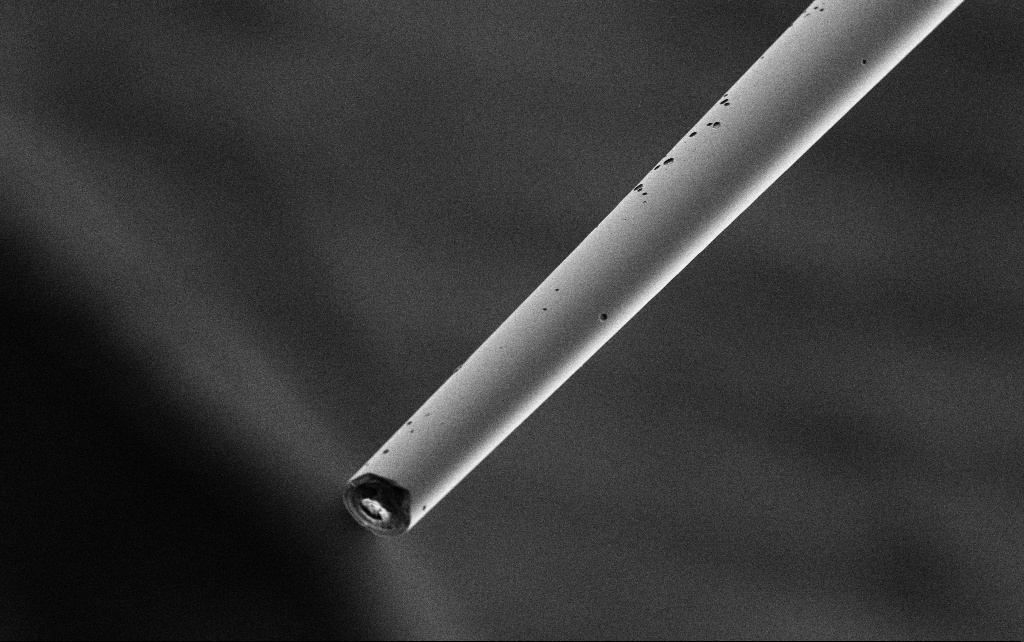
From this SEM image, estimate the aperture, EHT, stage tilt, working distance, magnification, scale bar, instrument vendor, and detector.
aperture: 30 µm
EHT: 3 kV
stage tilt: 45°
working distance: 7.8 mm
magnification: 1 K X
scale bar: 20000 nm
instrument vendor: Zeiss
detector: SE2